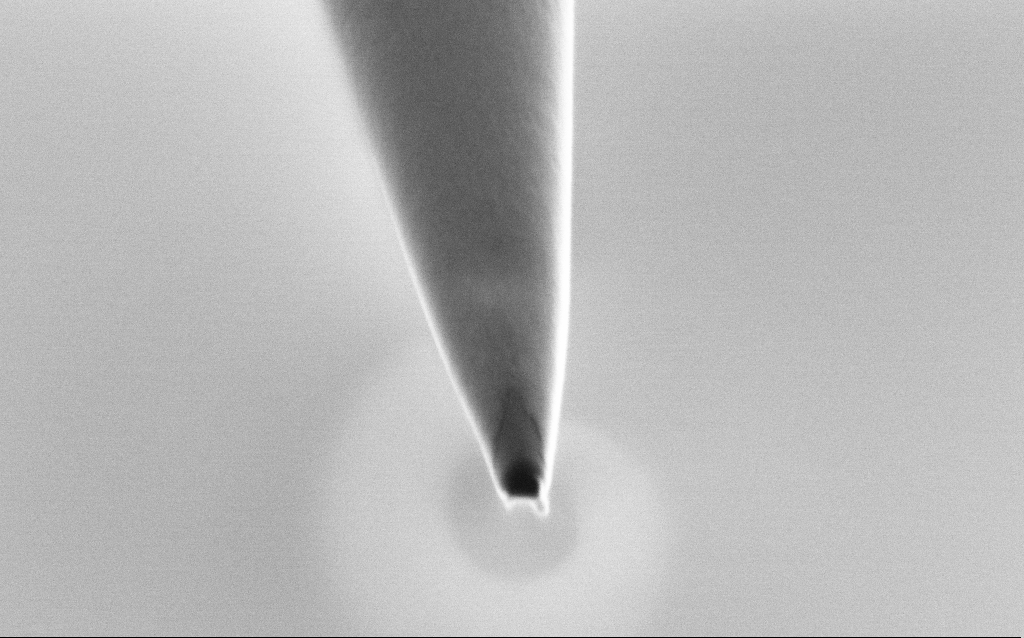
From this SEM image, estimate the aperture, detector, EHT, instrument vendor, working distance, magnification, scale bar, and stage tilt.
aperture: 30 µm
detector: SE2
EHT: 1 kV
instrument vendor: Zeiss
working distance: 6 mm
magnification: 99.65 K X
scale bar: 200 nm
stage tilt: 45°